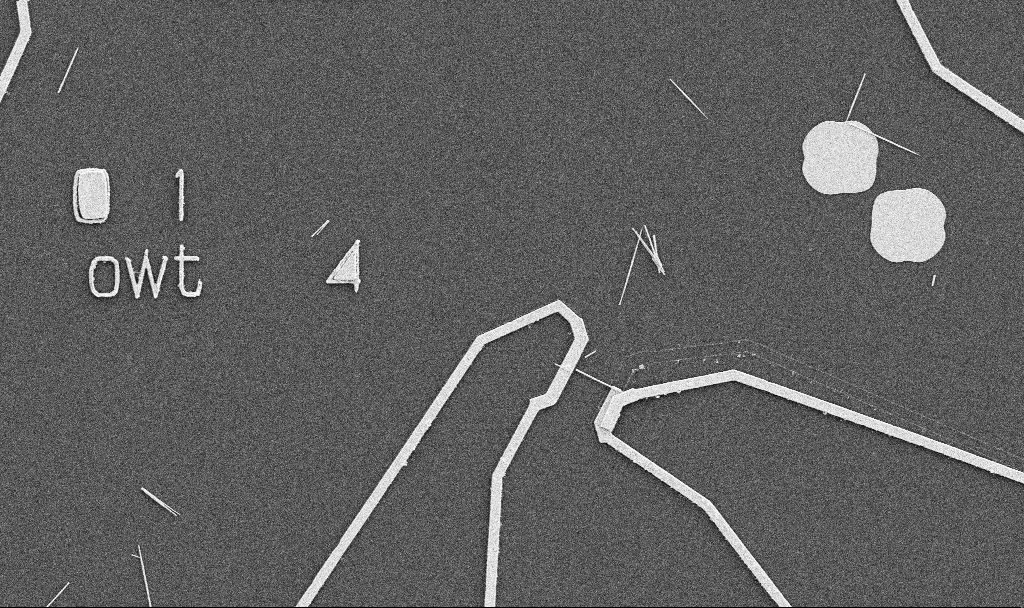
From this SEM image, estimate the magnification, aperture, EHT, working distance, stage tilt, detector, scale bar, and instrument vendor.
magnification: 5 K X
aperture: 30 µm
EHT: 5 kV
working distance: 10.7 mm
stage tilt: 0°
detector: SE2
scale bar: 10000 nm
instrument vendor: Zeiss